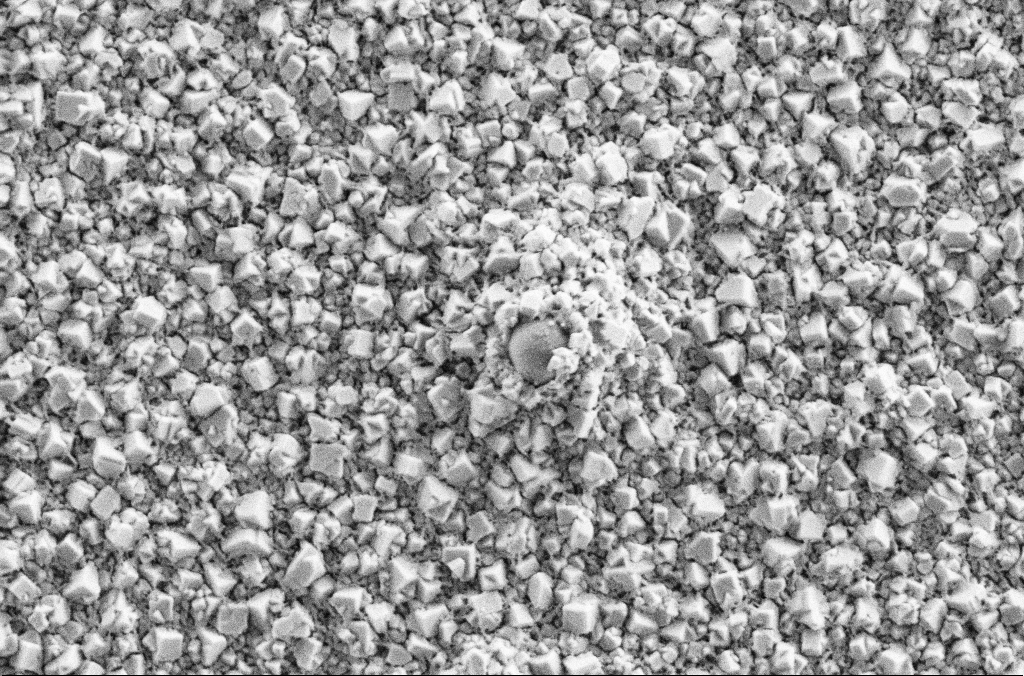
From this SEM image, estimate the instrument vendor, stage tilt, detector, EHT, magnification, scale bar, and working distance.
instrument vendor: Zeiss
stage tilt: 0°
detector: SE2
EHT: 2 kV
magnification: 30 K X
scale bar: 2000 nm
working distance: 1.9 mm